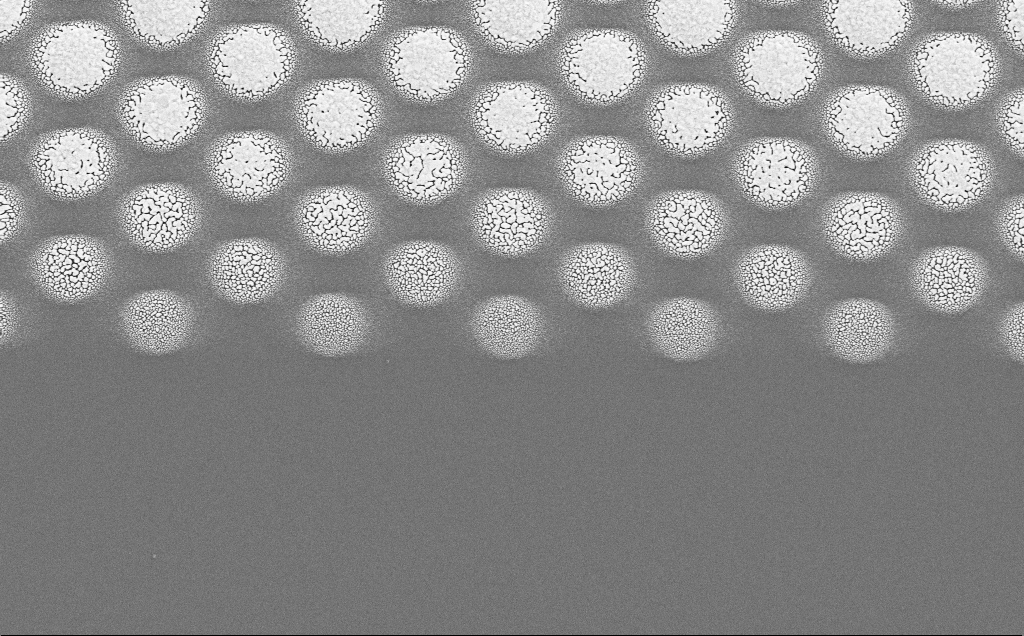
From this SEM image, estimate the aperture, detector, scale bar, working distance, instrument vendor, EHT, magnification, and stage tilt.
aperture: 30 µm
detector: SE2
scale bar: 2000 nm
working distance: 20 mm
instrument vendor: Zeiss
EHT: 5 kV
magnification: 12.29 K X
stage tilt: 0°